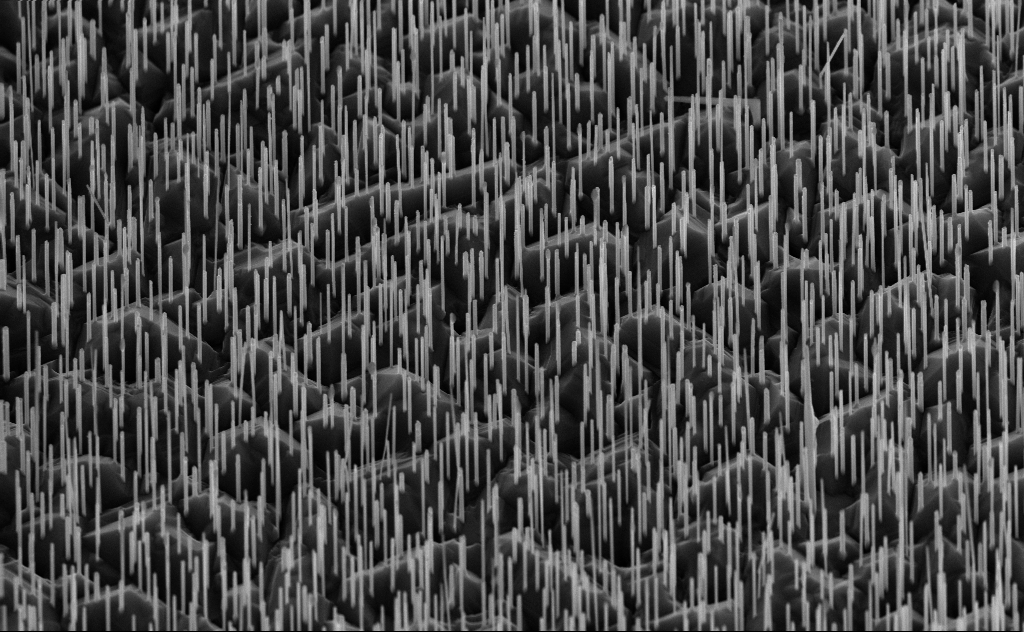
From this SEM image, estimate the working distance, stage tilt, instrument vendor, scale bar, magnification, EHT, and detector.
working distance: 6 mm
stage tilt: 45°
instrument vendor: Zeiss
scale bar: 2000 nm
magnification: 20 K X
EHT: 10 kV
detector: InLens